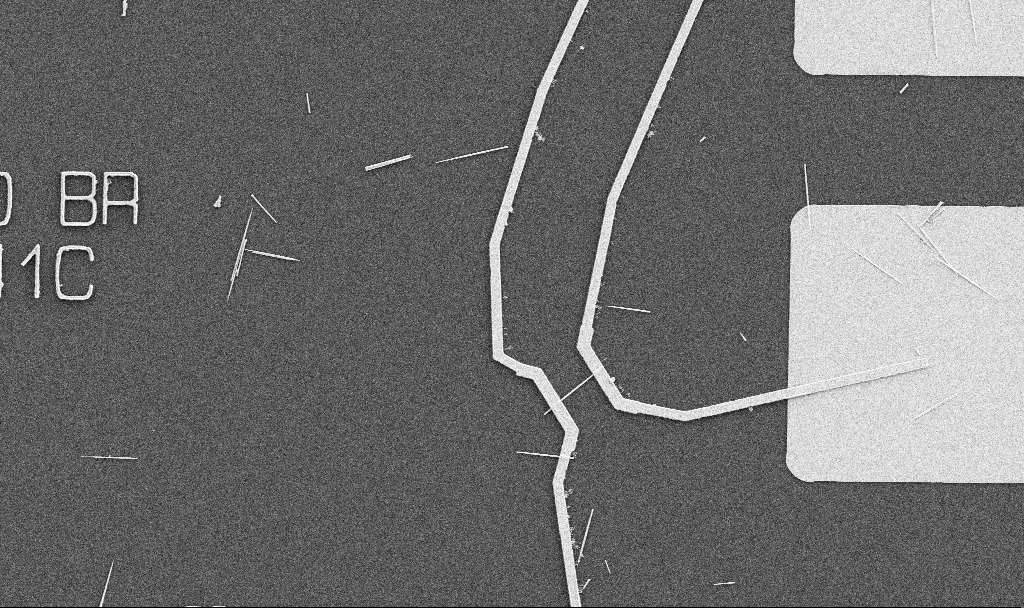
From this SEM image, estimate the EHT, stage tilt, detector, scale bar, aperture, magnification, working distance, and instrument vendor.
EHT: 5 kV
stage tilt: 0°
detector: SE2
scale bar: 10000 nm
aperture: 30 µm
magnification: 5 K X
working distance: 10.7 mm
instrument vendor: Zeiss